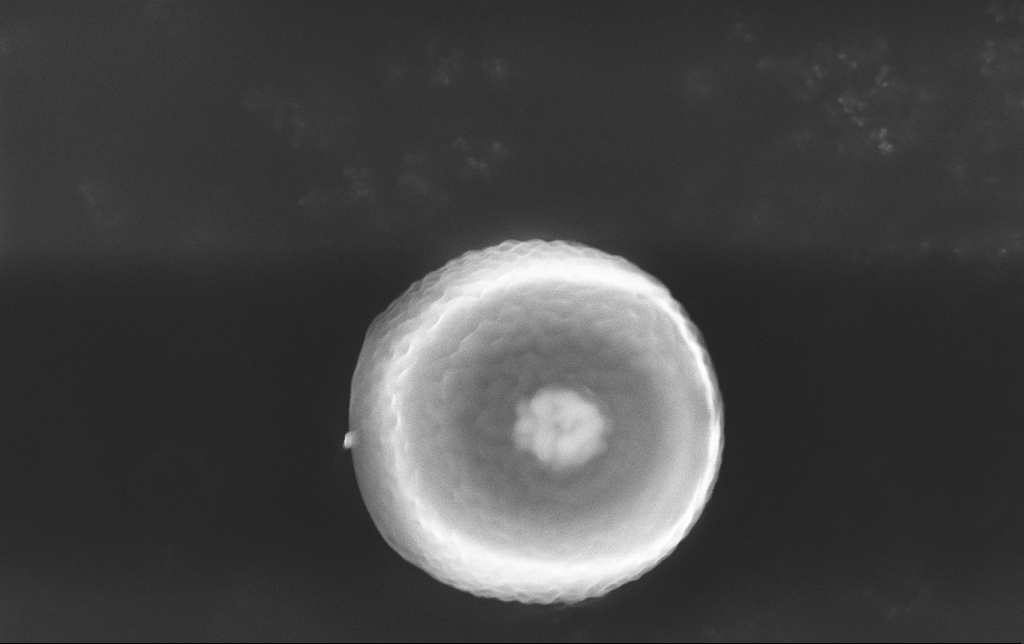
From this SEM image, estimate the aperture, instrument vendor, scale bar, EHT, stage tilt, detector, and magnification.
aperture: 30 µm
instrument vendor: Zeiss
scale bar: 2000 nm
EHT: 15 kV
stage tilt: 0°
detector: InLens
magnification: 20.31 K X